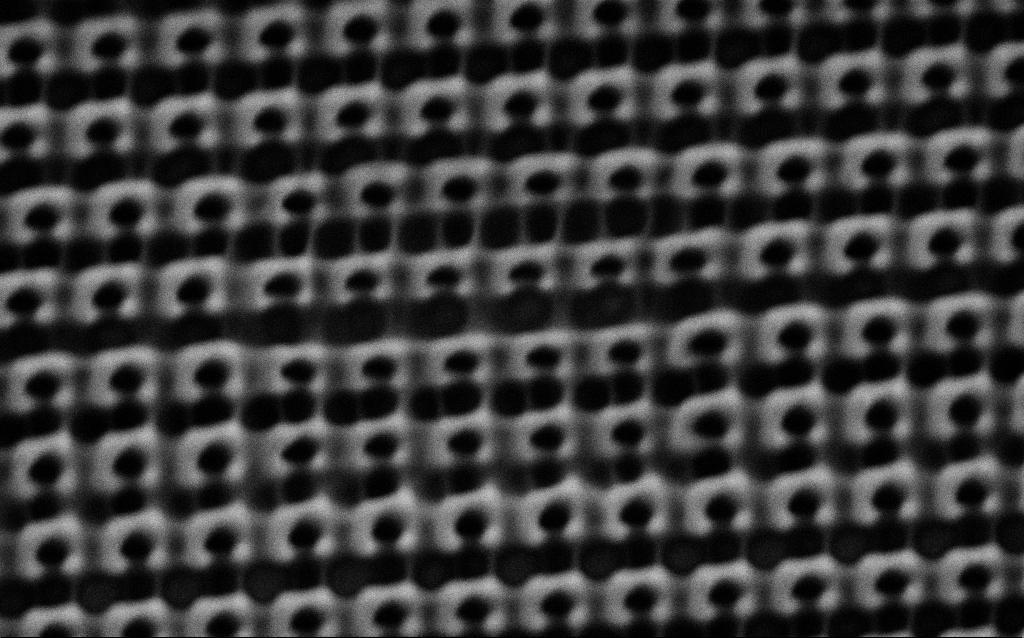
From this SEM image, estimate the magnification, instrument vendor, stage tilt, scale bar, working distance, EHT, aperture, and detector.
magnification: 65.97 K X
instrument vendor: Zeiss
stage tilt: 0°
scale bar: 1000 nm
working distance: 4.9 mm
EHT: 1.5 kV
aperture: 30 µm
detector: SE2